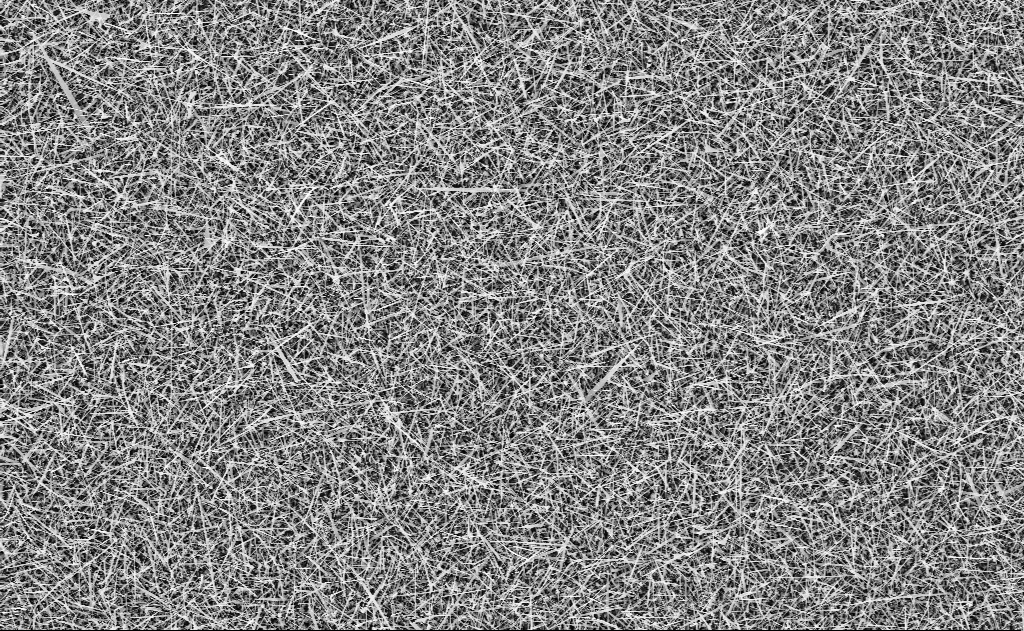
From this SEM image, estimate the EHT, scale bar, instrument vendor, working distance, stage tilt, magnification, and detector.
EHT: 10 kV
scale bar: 10000 nm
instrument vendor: Zeiss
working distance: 11 mm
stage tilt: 0°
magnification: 5 K X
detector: InLens